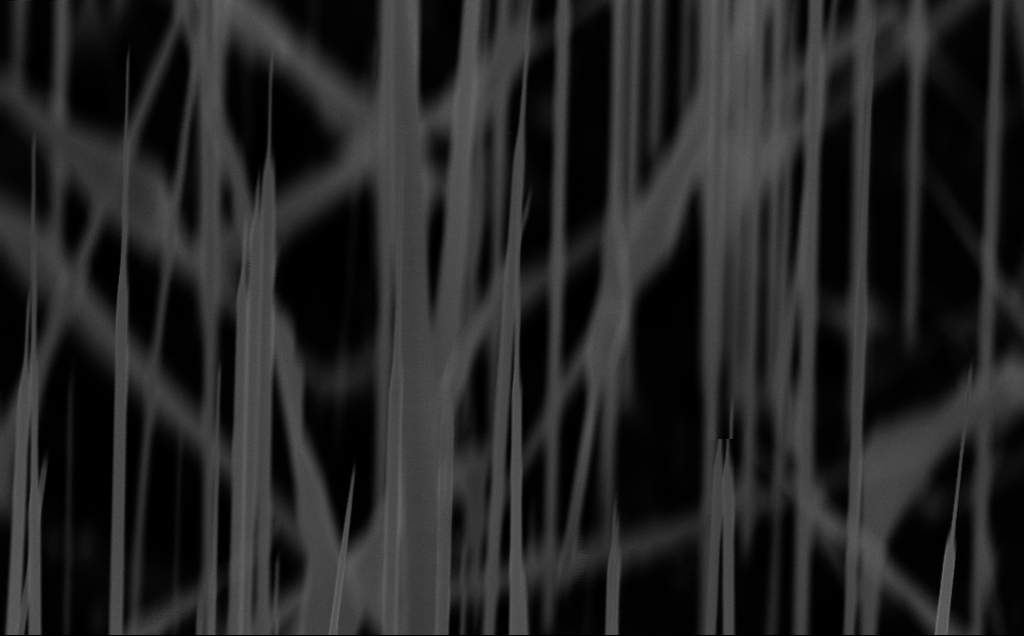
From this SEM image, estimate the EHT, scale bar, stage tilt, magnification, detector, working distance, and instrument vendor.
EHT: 10 kV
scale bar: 1000 nm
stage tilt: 45°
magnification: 56.44 K X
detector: InLens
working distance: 6 mm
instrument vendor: Zeiss